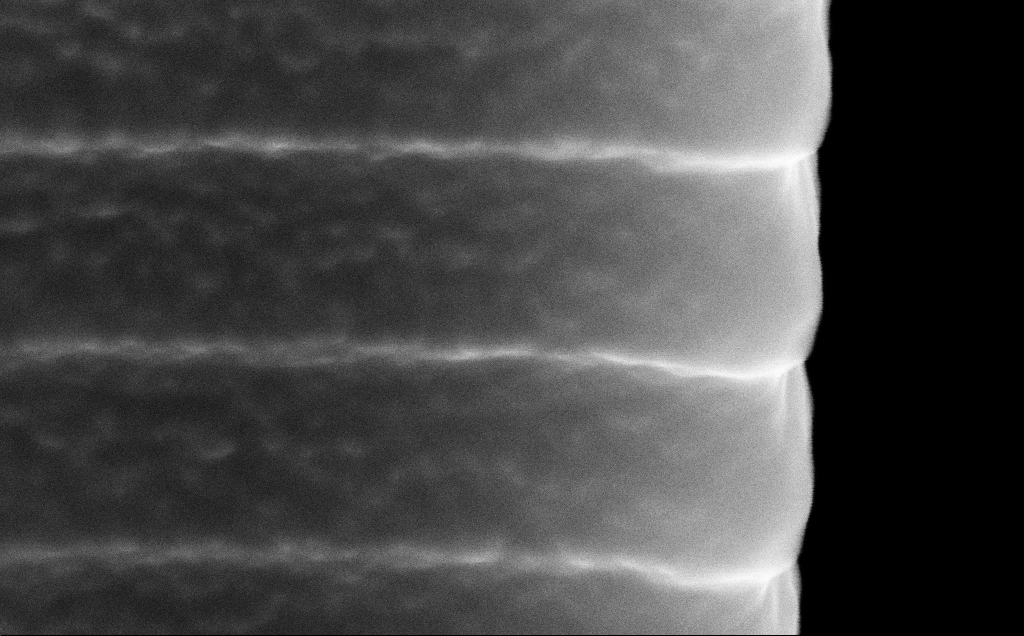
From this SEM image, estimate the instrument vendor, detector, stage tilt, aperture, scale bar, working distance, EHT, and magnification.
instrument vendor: Zeiss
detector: InLens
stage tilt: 45°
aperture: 30 µm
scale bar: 100 nm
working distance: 5 mm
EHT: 10 kV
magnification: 207.32 K X